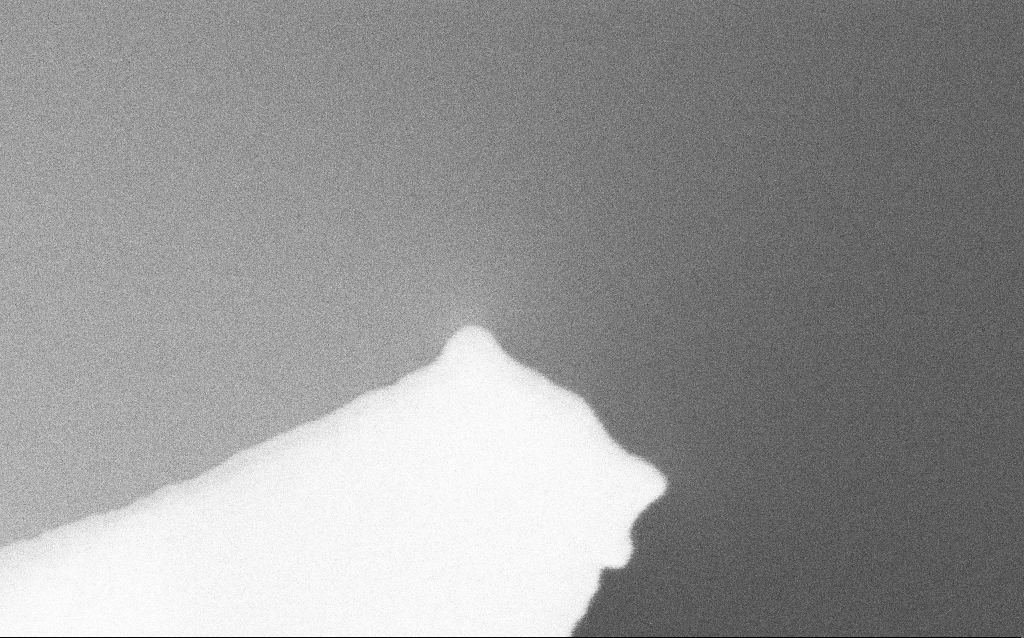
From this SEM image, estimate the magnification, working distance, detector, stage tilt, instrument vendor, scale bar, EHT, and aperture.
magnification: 518.85 K X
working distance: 2.9 mm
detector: InLens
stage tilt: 0°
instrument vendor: Zeiss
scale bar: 100 nm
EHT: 5 kV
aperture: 30 µm